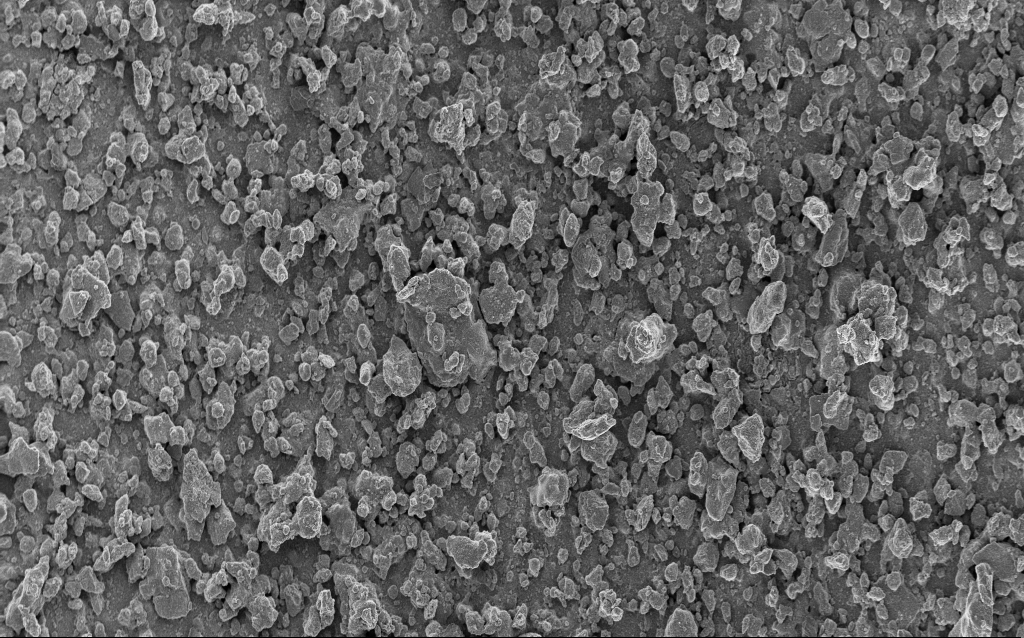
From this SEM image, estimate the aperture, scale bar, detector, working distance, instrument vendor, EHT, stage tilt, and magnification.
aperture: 30 µm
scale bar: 100000 nm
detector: InLens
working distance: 4.5 mm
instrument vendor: Zeiss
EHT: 5 kV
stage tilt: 0°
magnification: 0.581 K X